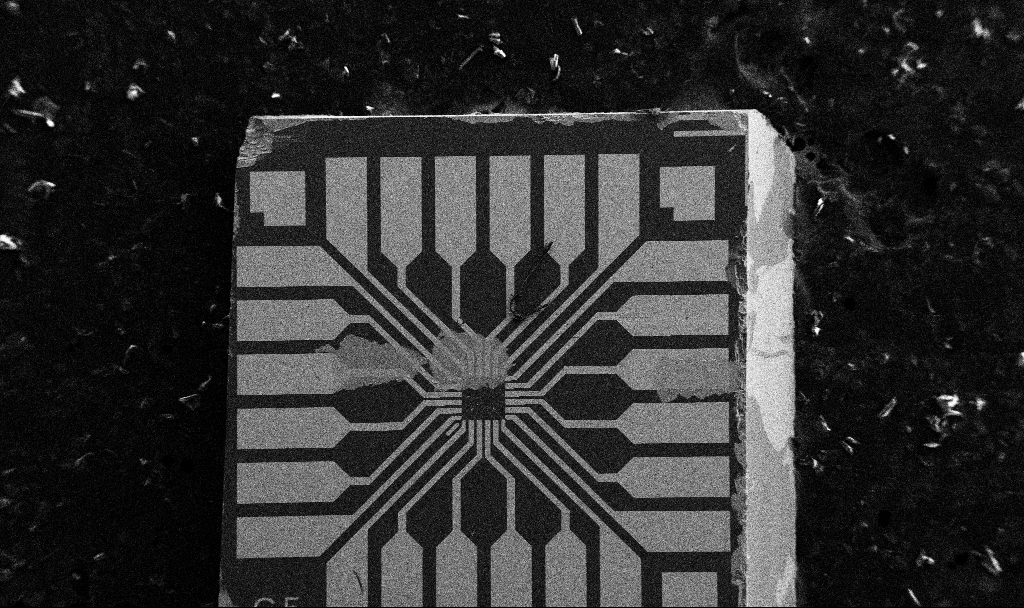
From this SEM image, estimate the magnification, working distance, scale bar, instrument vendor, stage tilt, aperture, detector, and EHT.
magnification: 0.1 K X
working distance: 10.7 mm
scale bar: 200000 nm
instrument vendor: Zeiss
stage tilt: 0°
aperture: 30 µm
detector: SE2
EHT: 5 kV